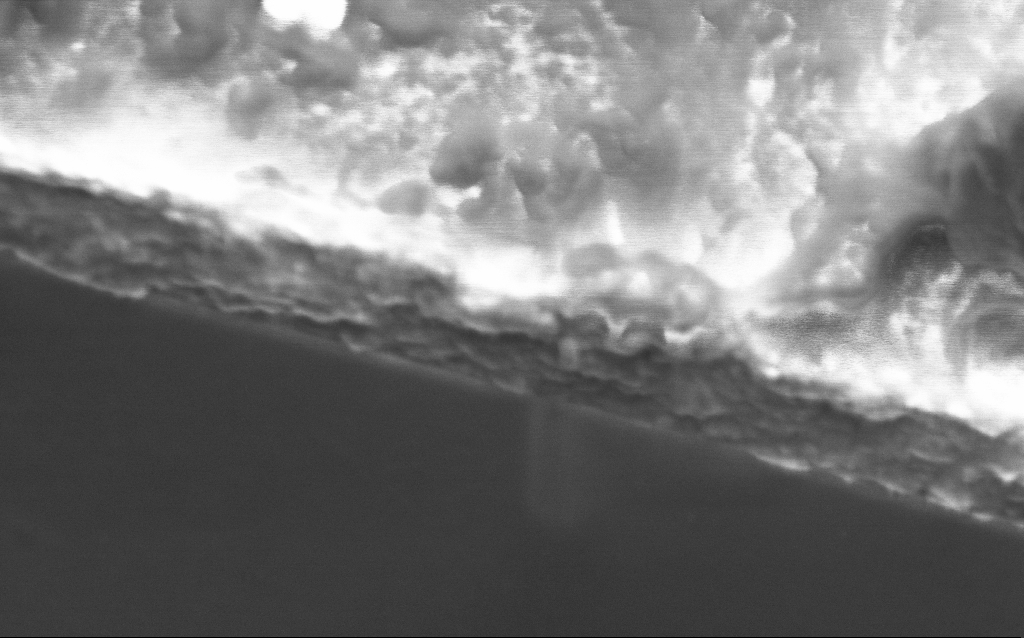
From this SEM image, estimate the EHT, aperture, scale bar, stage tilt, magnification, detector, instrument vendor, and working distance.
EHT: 1 kV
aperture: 30 µm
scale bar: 200 nm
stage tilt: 45°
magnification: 132.05 K X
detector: InLens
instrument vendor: Zeiss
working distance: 5 mm